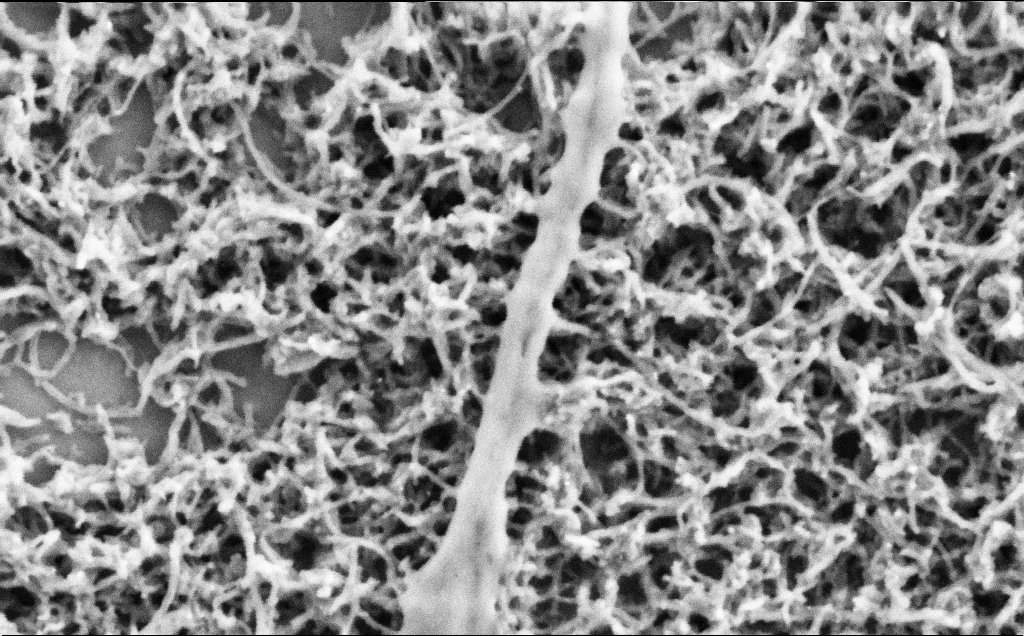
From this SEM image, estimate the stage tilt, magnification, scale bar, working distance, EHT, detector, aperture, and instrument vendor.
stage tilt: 0°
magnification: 80 K X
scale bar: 200 nm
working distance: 7.1 mm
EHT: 2 kV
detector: SE2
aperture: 30 µm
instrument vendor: Zeiss